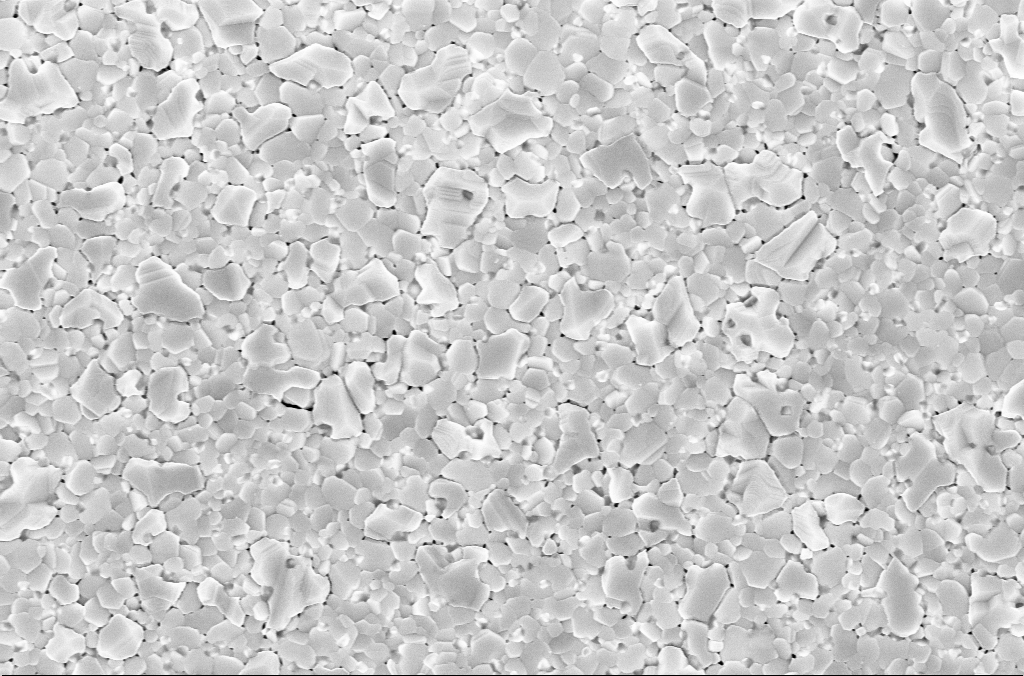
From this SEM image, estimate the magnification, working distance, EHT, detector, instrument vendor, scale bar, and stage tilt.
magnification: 40 K X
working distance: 3 mm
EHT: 5 kV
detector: InLens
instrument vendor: Zeiss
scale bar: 1000 nm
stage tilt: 0°